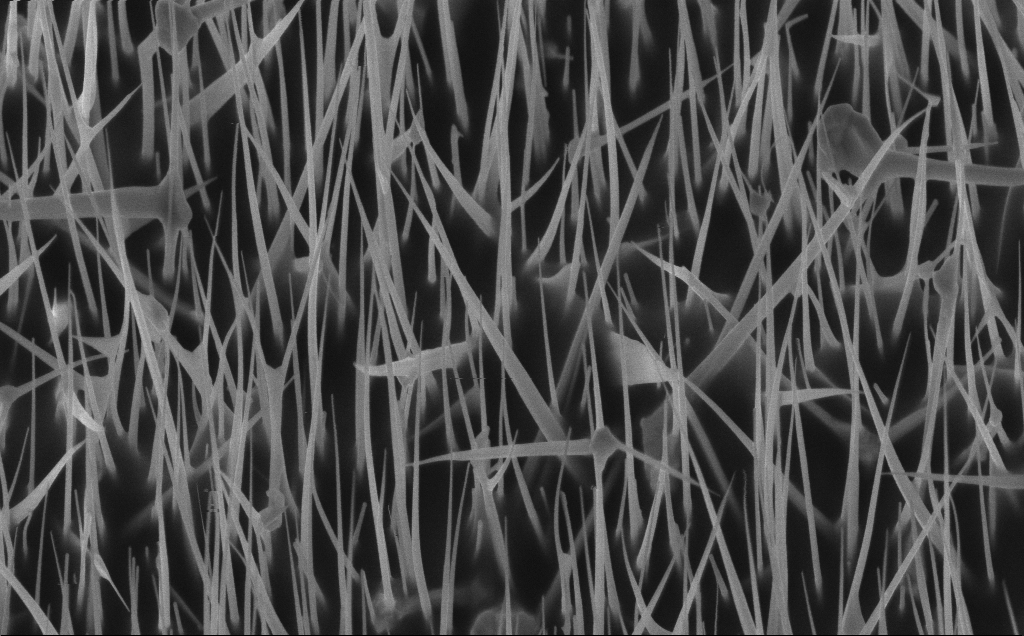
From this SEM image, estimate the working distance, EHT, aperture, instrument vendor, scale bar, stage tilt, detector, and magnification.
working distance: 7 mm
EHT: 5 kV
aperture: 30 µm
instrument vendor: Zeiss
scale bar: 2000 nm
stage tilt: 30°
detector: InLens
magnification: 32.75 K X